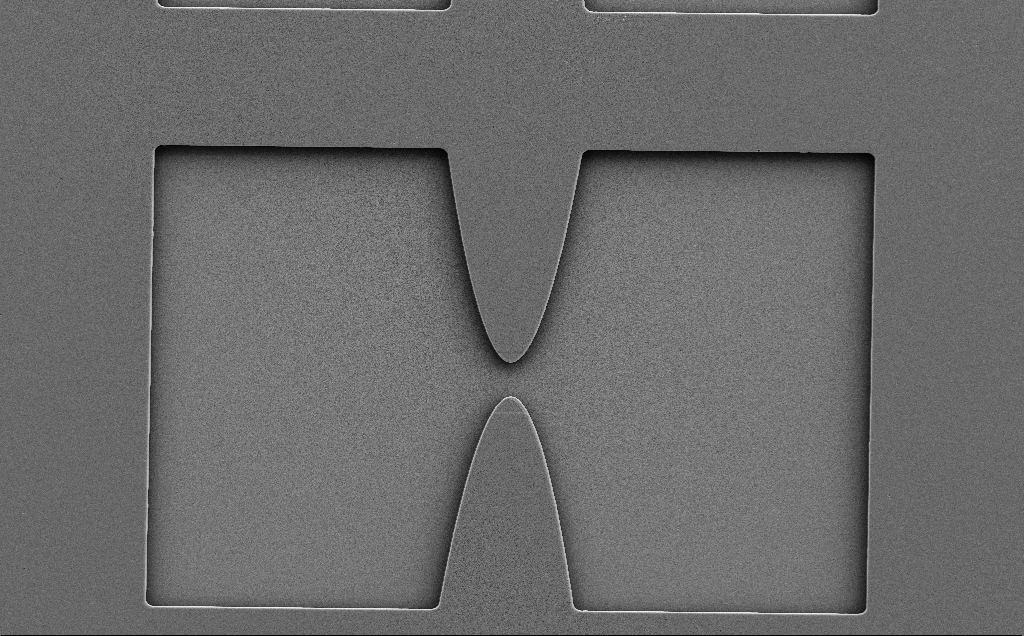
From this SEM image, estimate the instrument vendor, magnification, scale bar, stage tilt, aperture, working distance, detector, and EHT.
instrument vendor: Zeiss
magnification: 1.06 K X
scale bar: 20000 nm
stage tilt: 0°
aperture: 30 µm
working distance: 9 mm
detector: SE2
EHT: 5 kV